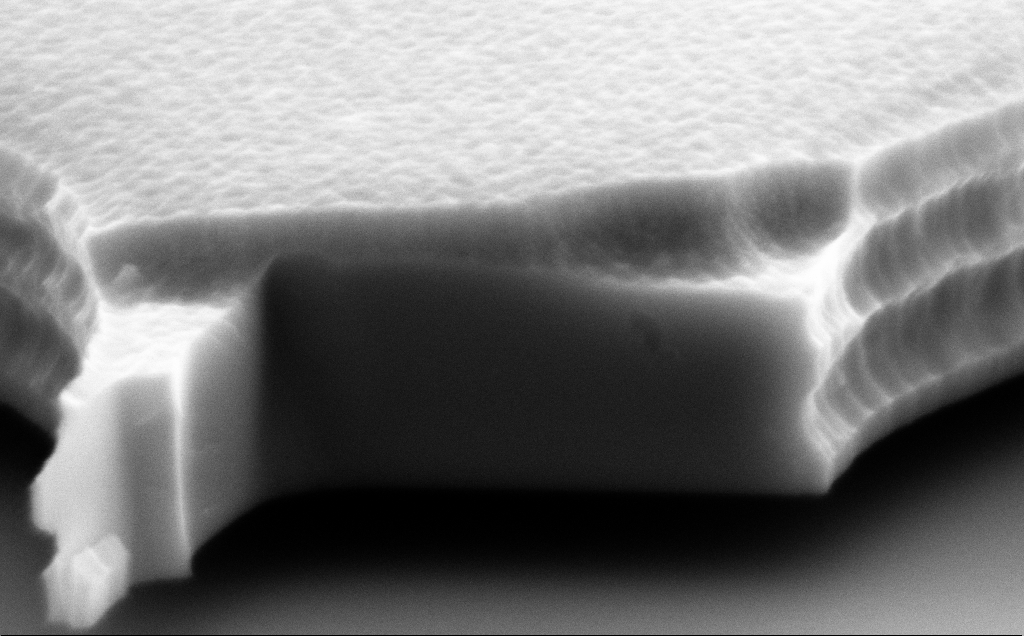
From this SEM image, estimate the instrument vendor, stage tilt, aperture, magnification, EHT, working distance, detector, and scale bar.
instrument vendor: Zeiss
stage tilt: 70°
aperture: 30 µm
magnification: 52 K X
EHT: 8 kV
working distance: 12 mm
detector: SE2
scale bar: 1000 nm